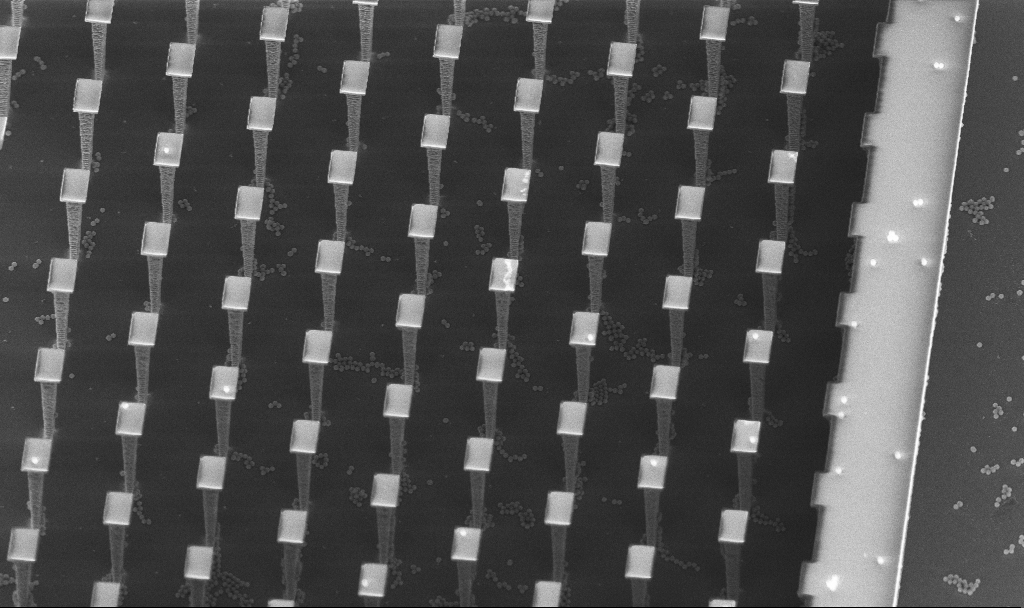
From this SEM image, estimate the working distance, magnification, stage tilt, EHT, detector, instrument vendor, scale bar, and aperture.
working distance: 5.1 mm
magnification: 3.2 K X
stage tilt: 30°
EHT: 5 kV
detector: InLens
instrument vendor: Zeiss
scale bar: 10000 nm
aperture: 30 µm